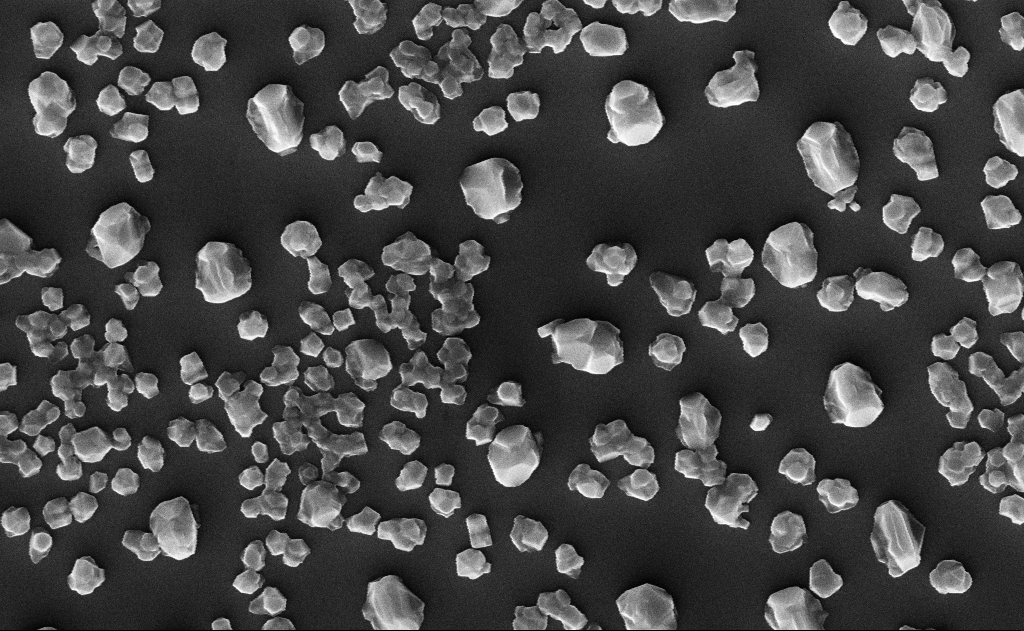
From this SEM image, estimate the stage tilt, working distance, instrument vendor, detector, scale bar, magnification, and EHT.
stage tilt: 0°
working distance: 18 mm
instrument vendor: Zeiss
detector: InLens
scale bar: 1000 nm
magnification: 40 K X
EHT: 10 kV